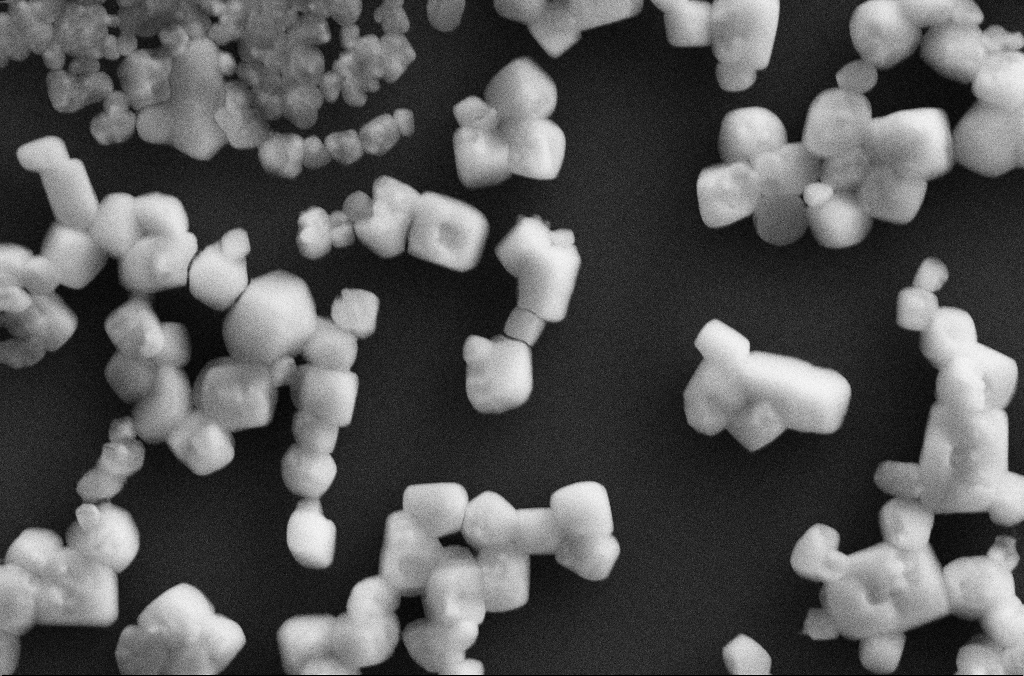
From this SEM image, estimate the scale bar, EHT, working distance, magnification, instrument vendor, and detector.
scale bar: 2000 nm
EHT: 20 kV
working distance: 2.8 mm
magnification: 10 K X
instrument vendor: Zeiss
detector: SE2